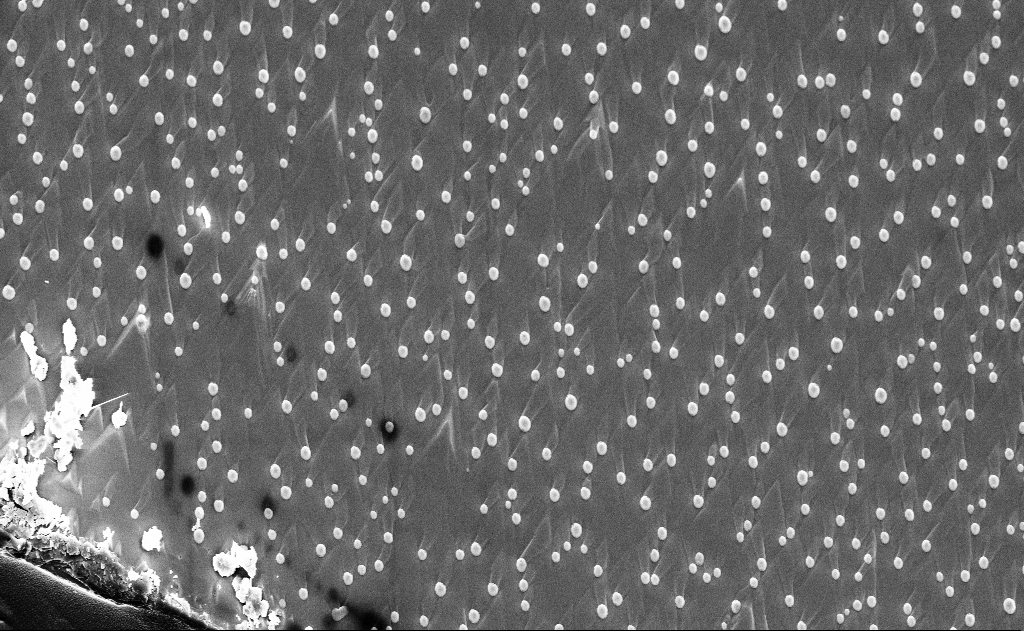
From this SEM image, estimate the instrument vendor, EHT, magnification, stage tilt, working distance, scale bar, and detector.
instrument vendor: Zeiss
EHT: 10 kV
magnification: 5 K X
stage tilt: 0°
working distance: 12 mm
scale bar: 10000 nm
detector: InLens